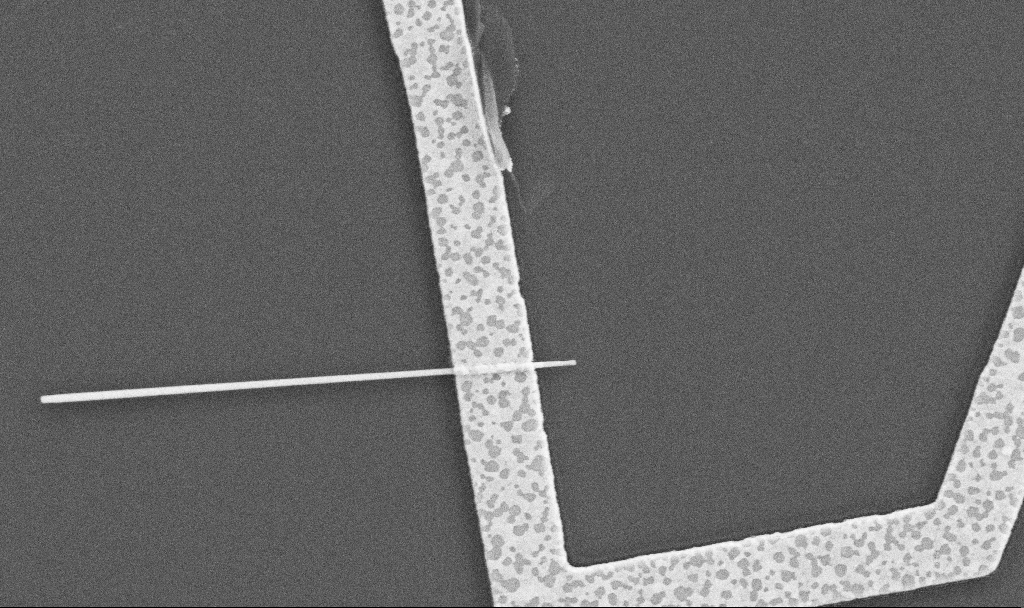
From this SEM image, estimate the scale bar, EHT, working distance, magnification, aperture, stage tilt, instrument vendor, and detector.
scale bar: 1000 nm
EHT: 5 kV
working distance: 10.5 mm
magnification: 30 K X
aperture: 30 µm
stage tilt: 0°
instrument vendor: Zeiss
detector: SE2